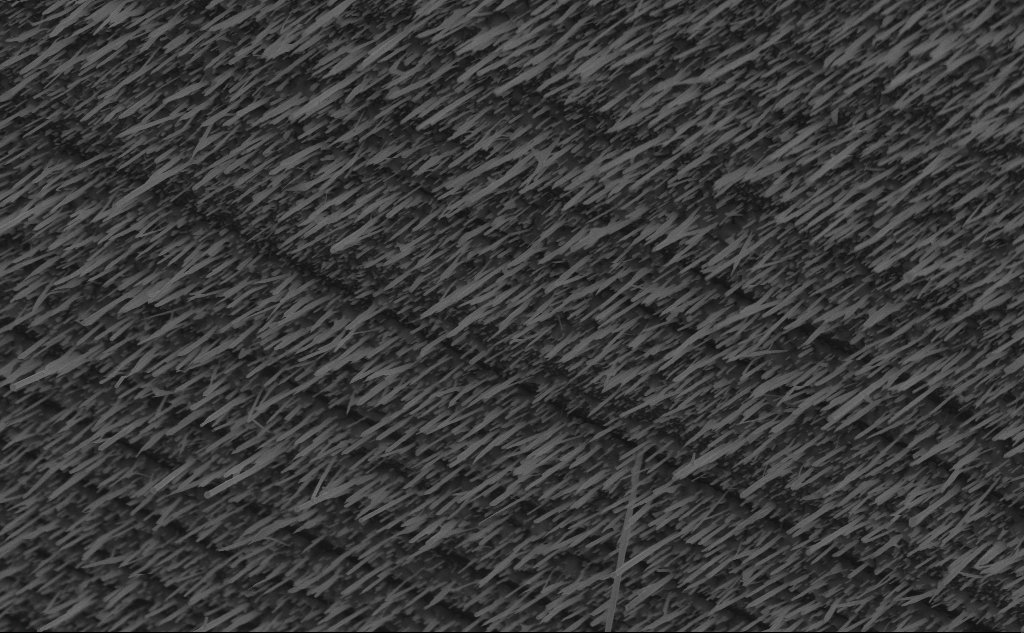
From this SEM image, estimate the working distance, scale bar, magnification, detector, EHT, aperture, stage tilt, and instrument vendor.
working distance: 5 mm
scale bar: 2000 nm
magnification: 22.07 K X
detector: InLens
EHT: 10 kV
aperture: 30 µm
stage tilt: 45°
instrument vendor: Zeiss